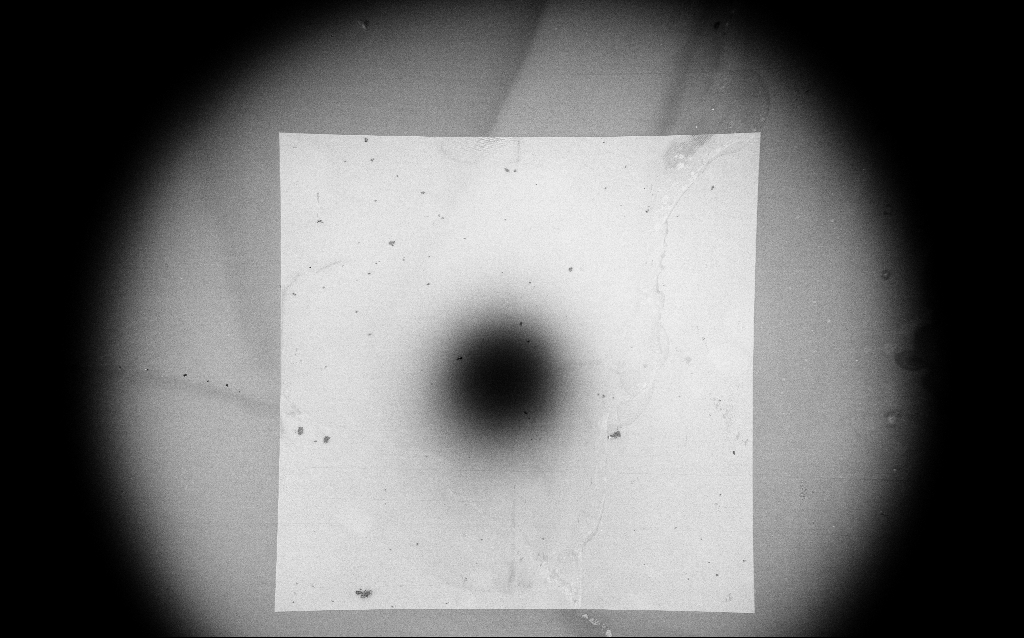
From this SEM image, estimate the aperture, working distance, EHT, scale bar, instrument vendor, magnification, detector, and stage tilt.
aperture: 30 µm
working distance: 2.9 mm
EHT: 3 kV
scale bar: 200000 nm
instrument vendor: Zeiss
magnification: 0.087 K X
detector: InLens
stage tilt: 0°